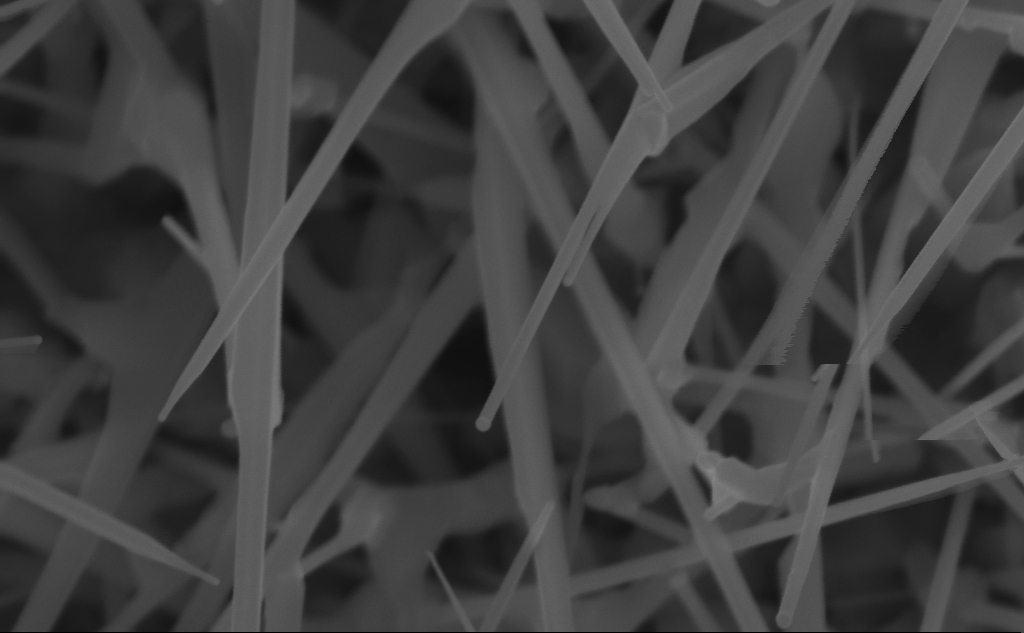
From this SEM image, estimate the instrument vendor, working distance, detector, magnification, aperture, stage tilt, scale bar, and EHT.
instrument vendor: Zeiss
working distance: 6 mm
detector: InLens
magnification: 141.72 K X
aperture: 30 µm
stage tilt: -0.2°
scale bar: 200 nm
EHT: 10 kV